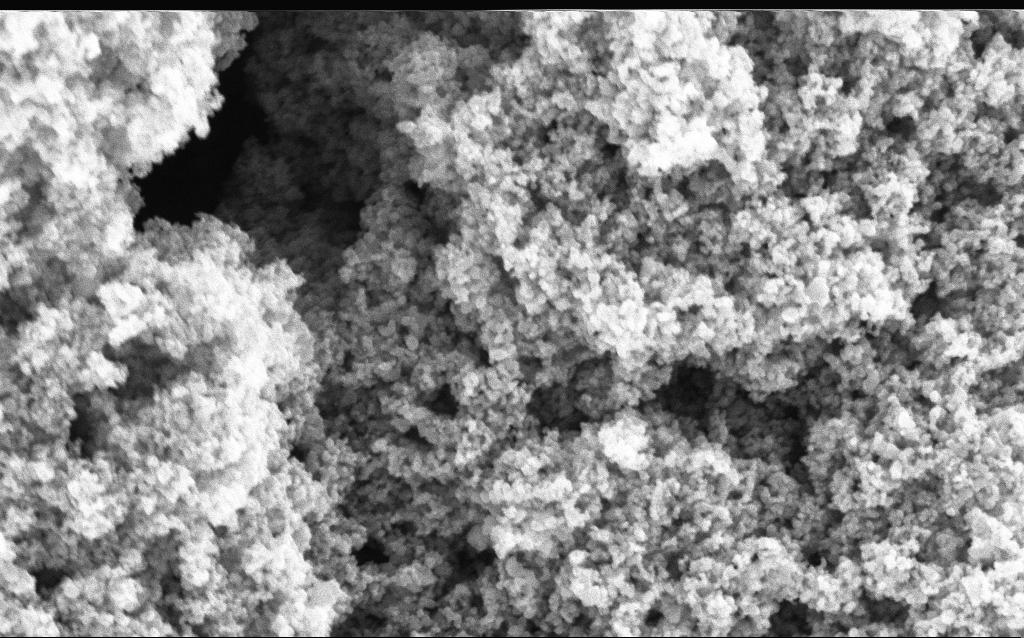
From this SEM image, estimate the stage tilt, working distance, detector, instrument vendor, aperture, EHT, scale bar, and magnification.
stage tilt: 0°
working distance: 4.6 mm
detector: InLens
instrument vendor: Zeiss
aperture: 30 µm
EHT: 5 kV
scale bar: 200 nm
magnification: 88.07 K X